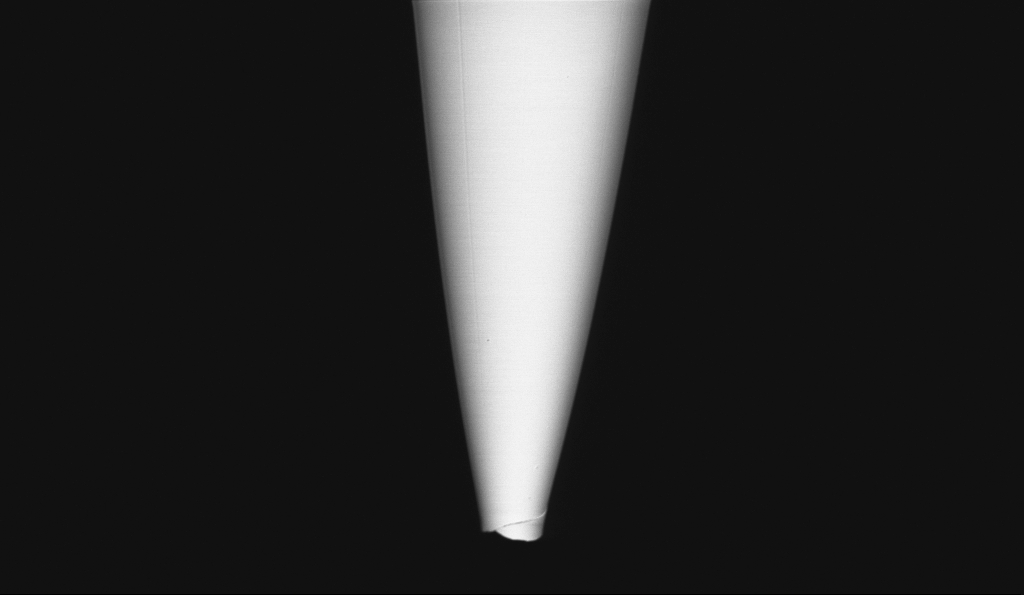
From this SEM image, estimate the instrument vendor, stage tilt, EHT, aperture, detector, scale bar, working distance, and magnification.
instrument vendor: Zeiss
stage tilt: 0°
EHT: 1 kV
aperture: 30 µm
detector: InLens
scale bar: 2000 nm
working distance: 5.7 mm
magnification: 10 K X